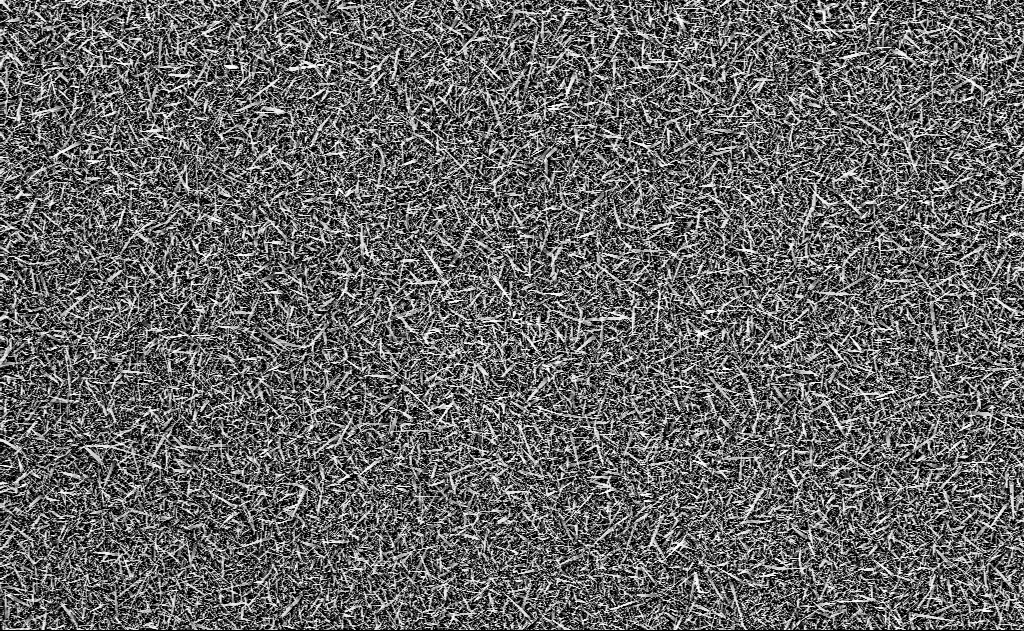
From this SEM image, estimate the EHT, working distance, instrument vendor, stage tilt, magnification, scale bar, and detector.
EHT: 10 kV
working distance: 12 mm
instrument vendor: Zeiss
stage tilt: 0°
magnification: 1 K X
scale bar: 20000 nm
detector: InLens